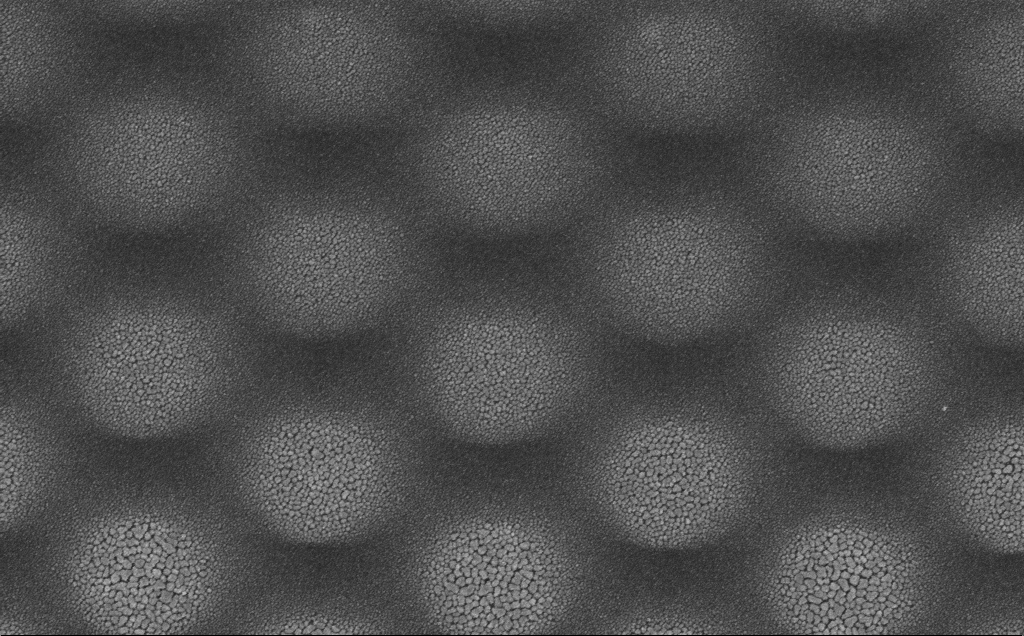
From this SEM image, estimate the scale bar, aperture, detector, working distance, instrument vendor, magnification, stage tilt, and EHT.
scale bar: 1000 nm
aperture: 30 µm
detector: InLens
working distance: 20 mm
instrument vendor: Zeiss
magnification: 24.49 K X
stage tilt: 0°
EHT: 5 kV